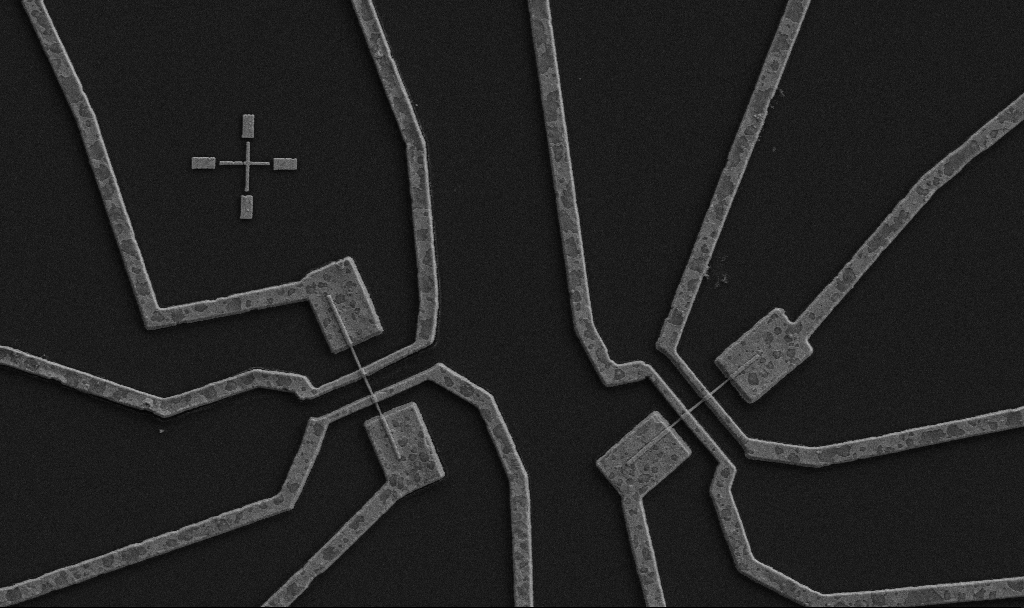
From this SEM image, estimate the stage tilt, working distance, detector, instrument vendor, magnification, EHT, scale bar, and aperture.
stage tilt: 0°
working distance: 9.7 mm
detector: SE2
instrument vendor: Zeiss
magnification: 10 K X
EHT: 5 kV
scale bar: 2000 nm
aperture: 30 µm